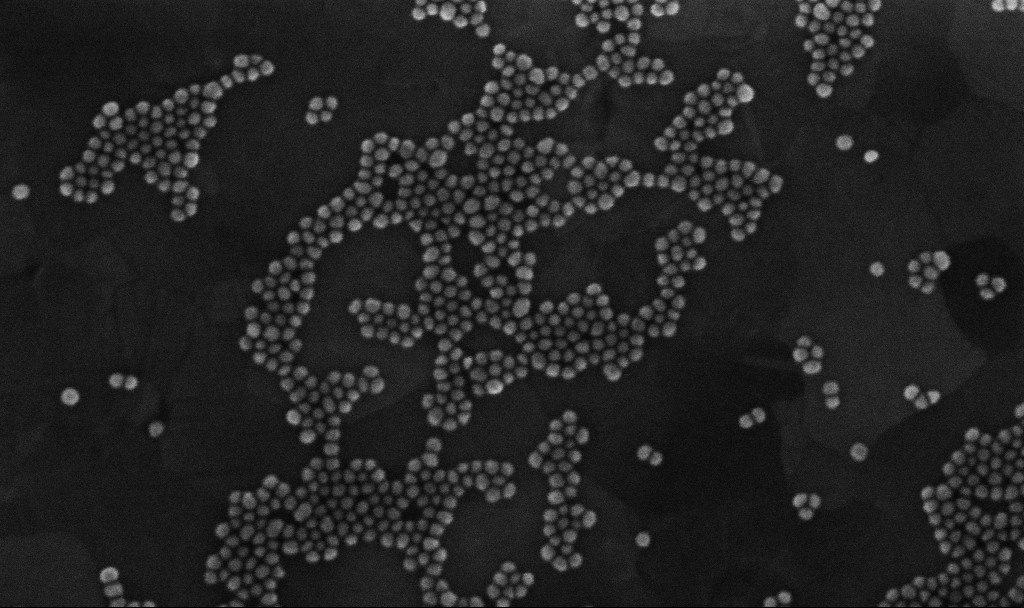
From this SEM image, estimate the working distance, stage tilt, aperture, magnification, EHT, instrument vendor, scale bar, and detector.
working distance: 3.4 mm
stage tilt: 0°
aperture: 30 µm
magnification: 300 K X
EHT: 10 kV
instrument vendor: Zeiss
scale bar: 100 nm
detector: InLens